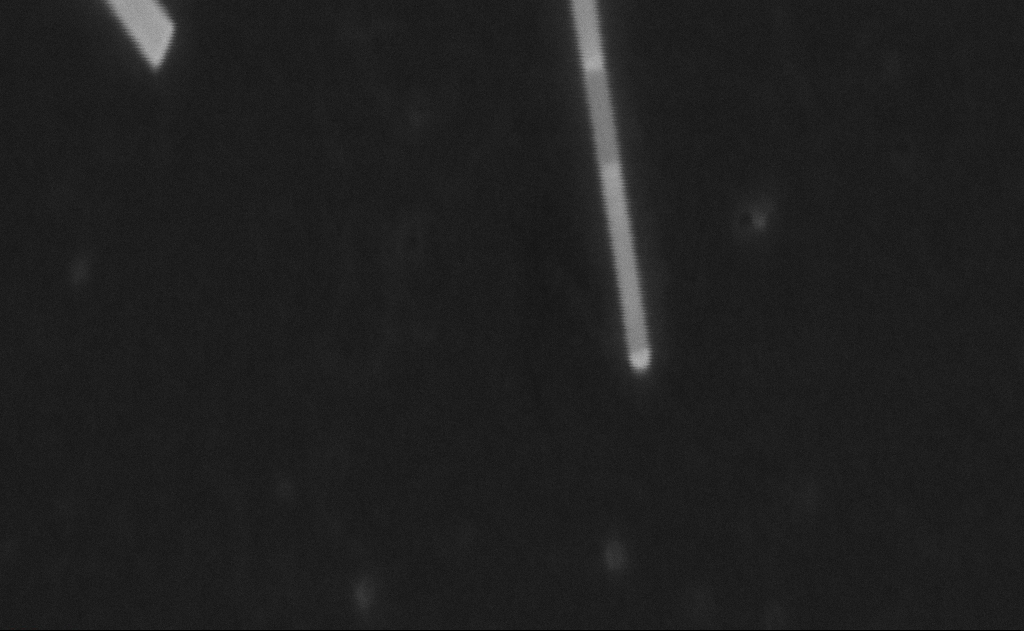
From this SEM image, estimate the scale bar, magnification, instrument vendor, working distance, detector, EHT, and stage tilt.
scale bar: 200 nm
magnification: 269.5 K X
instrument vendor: Zeiss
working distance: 16 mm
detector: SE2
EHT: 27 kV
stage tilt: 0°